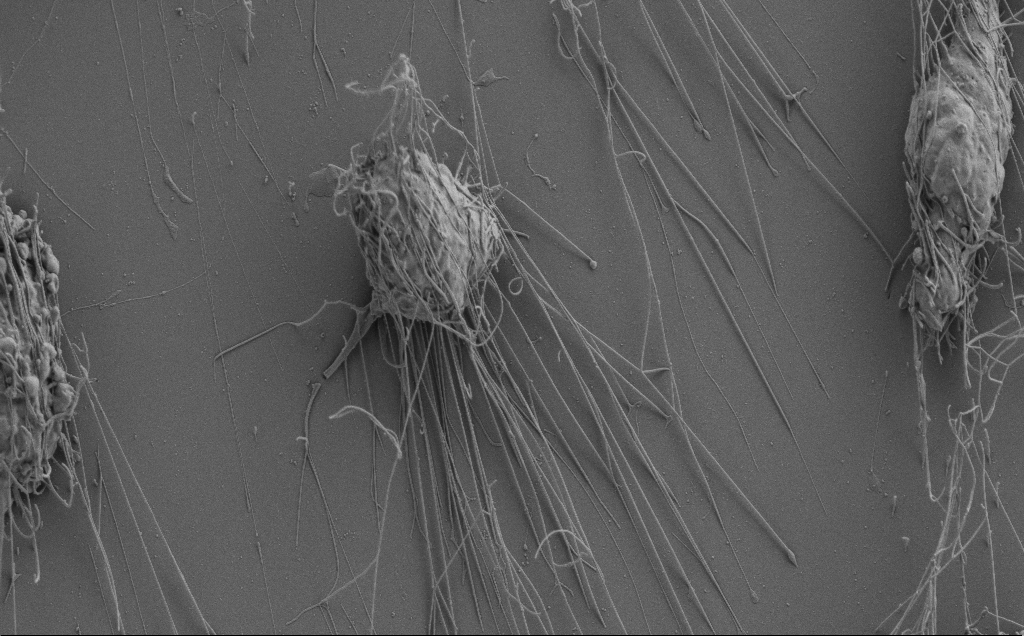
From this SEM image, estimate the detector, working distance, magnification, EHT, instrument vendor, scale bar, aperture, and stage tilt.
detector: SE2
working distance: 6 mm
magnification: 5.22 K X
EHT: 3 kV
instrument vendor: Zeiss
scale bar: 10000 nm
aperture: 30 µm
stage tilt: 0.8°